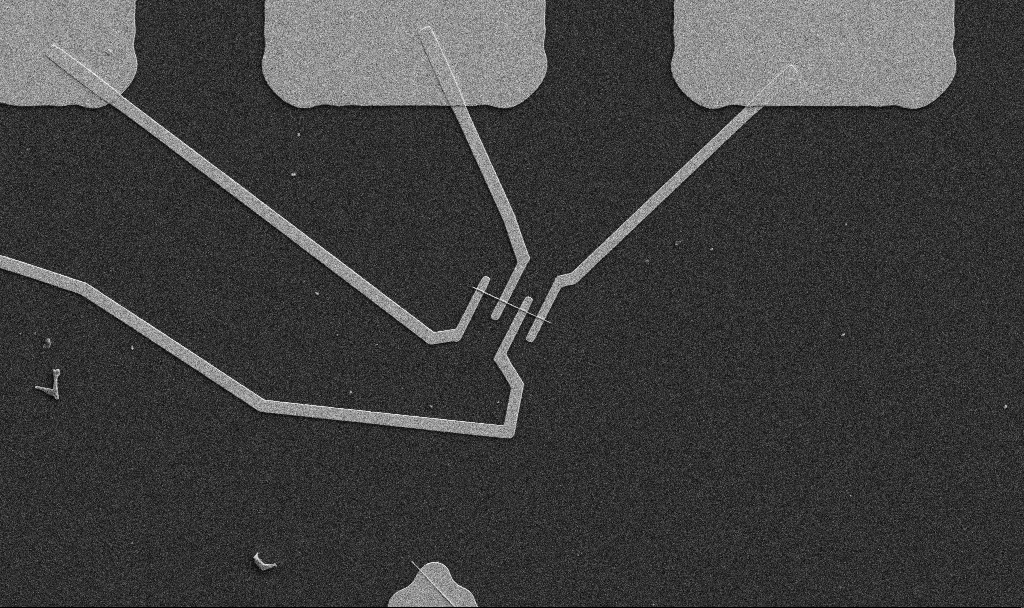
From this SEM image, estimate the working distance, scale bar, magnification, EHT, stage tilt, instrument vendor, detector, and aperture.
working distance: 10.7 mm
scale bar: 10000 nm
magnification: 5 K X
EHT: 5 kV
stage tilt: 0°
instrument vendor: Zeiss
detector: SE2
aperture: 30 µm